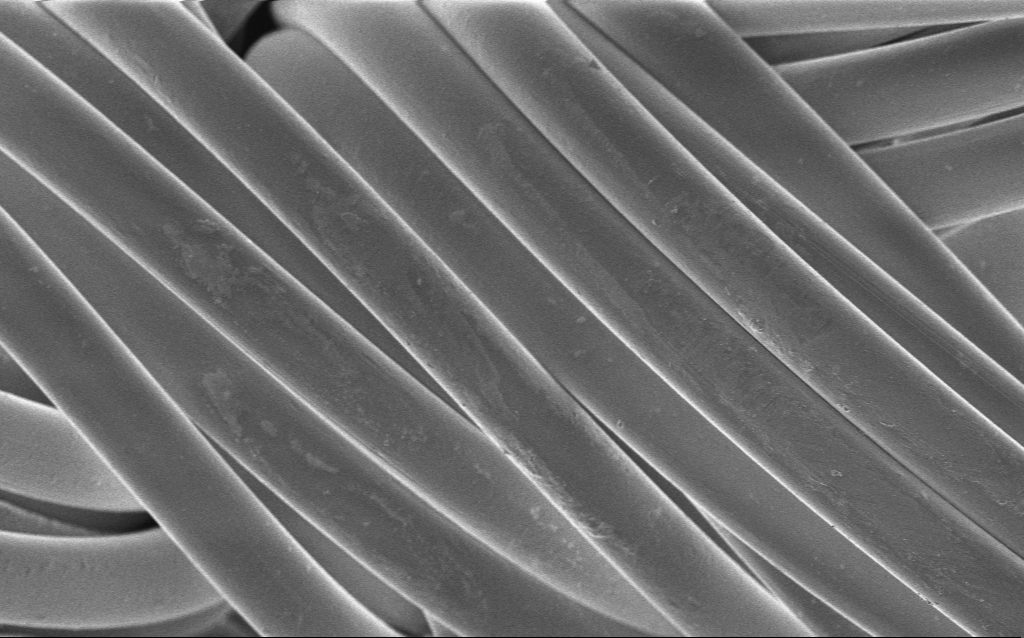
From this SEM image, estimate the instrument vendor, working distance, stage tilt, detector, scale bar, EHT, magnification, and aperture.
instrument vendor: Zeiss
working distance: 4 mm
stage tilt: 0°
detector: InLens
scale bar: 20000 nm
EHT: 1 kV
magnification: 1.43 K X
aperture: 30 µm